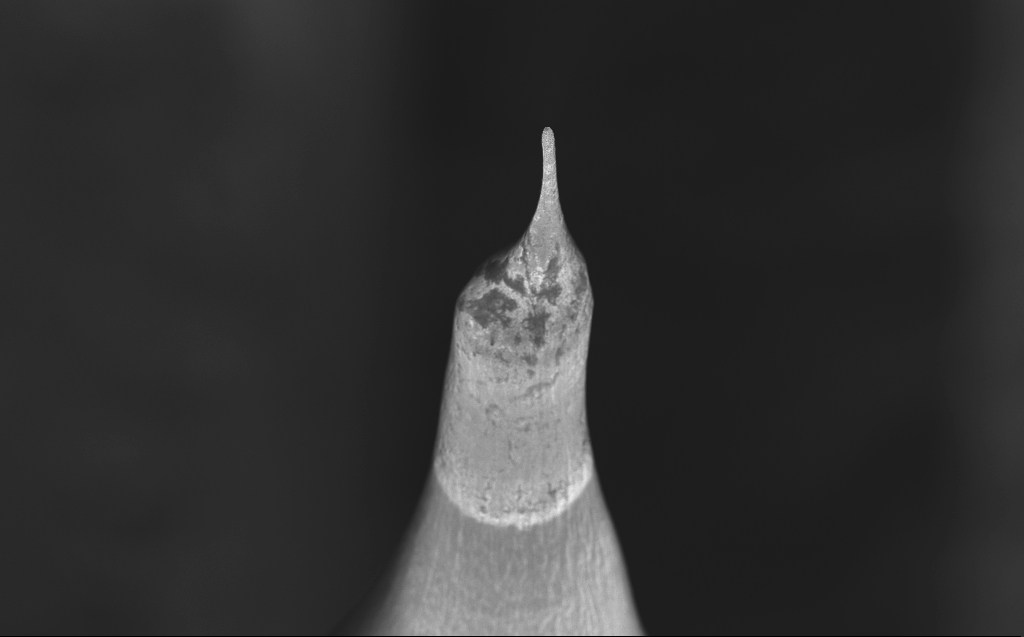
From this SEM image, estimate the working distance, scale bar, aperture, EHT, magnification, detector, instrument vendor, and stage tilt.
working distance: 4 mm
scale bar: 100000 nm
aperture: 30 µm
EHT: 10 kV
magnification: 0.623 K X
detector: InLens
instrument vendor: Zeiss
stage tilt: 40°